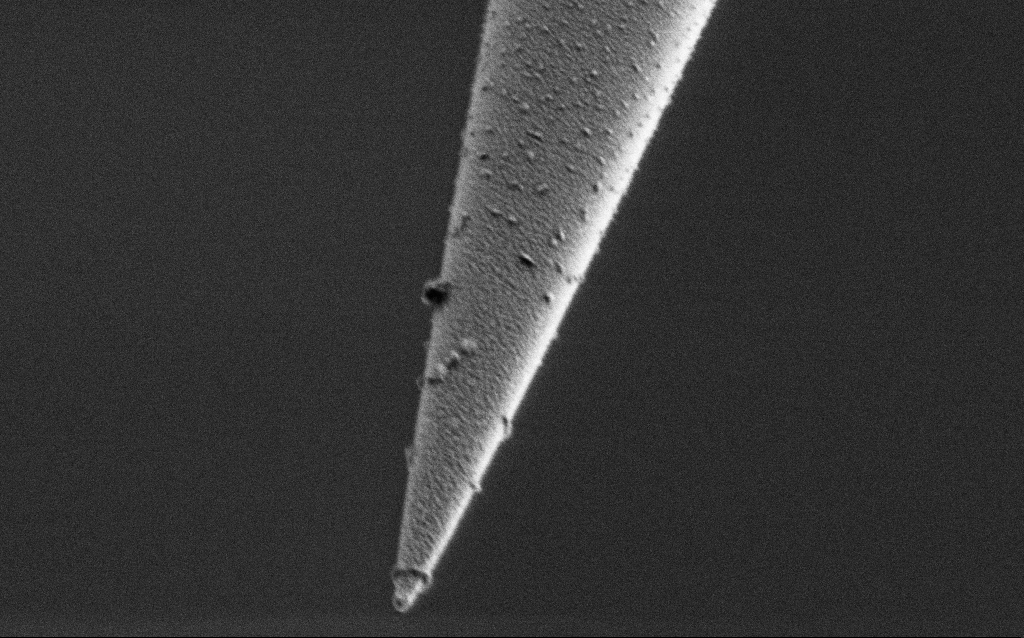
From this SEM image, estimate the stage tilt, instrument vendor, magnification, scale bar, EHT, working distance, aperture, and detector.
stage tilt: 45°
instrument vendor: Zeiss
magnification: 50 K X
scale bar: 1000 nm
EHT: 1 kV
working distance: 6.5 mm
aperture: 30 µm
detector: SE2